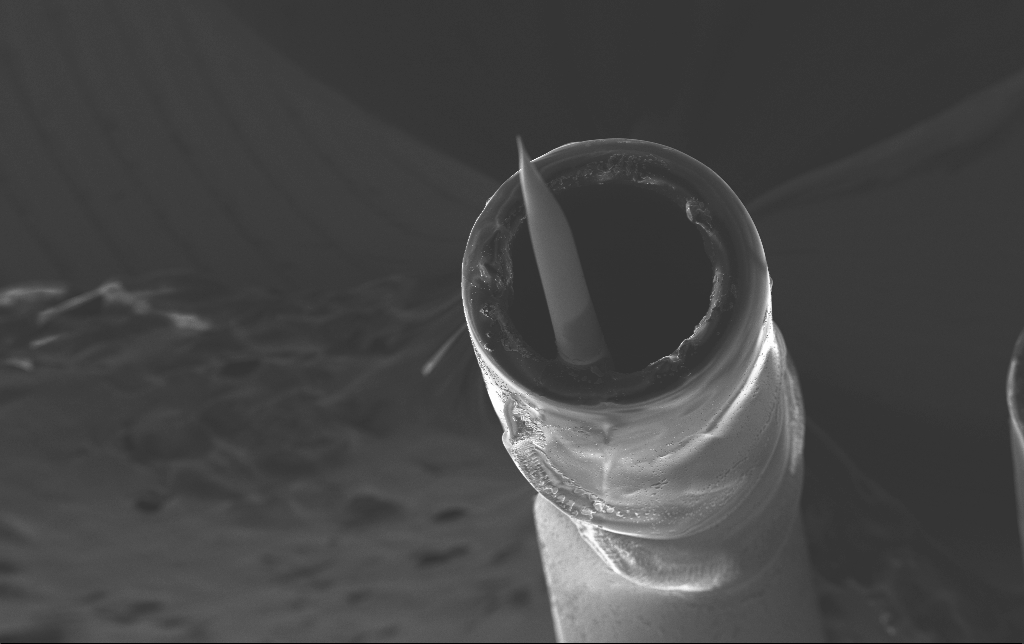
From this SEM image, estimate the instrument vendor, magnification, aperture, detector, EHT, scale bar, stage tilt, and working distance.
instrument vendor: Zeiss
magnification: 0.122 K X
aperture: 30 µm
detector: SE2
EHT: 10 kV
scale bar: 200000 nm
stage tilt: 69.3°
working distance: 9.1 mm